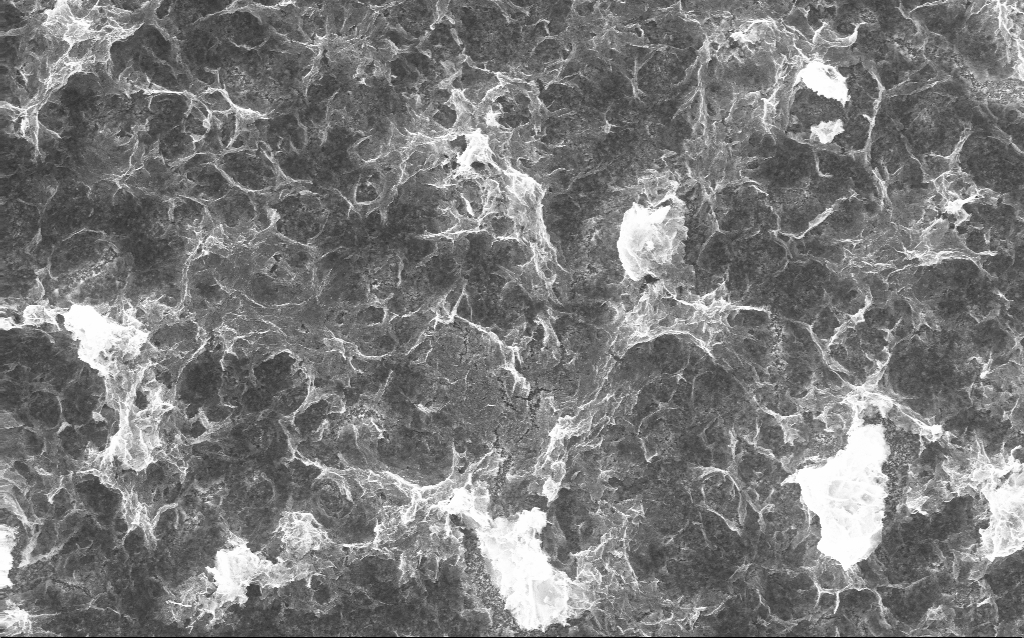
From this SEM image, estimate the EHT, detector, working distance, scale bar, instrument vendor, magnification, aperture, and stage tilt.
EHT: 10 kV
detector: InLens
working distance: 2.8 mm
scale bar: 10000 nm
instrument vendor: Zeiss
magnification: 5 K X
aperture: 30 µm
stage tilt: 0°